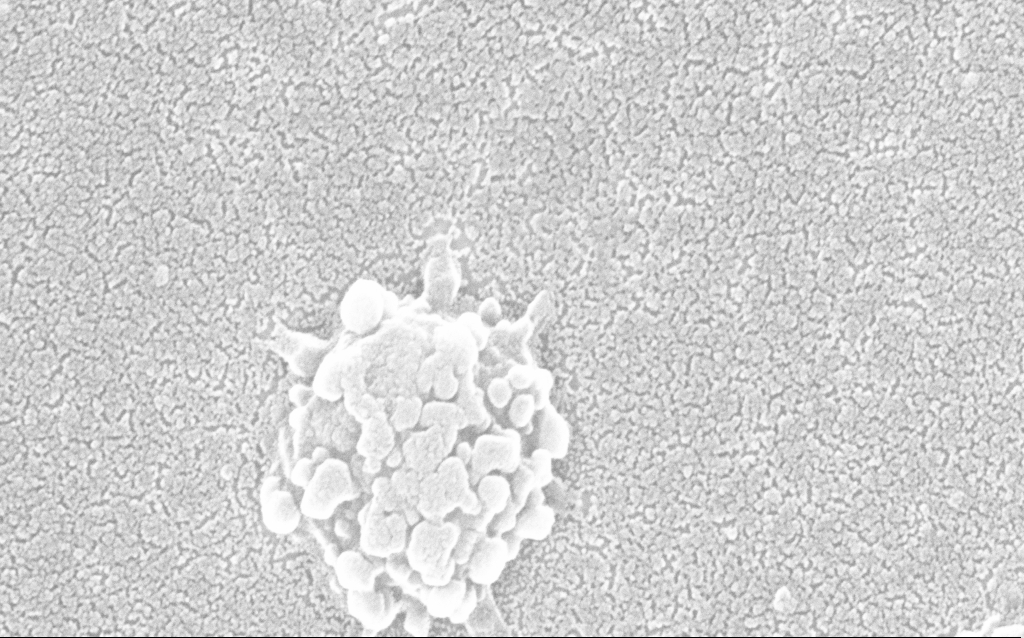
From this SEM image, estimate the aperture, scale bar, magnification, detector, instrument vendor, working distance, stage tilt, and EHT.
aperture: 30 µm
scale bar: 100 nm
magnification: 300 K X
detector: InLens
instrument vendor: Zeiss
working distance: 1.8 mm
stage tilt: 0°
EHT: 20 kV